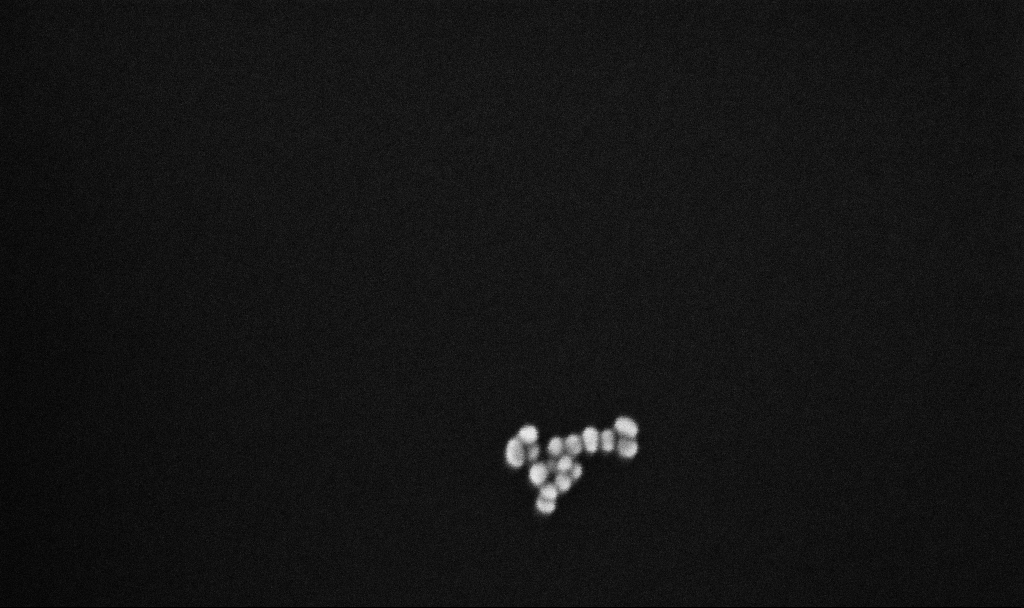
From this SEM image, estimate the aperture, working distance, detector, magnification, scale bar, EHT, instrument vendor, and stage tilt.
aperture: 30 µm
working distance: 5.4 mm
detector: InLens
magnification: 366.8 K X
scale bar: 100 nm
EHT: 10 kV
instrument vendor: Zeiss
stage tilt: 0°